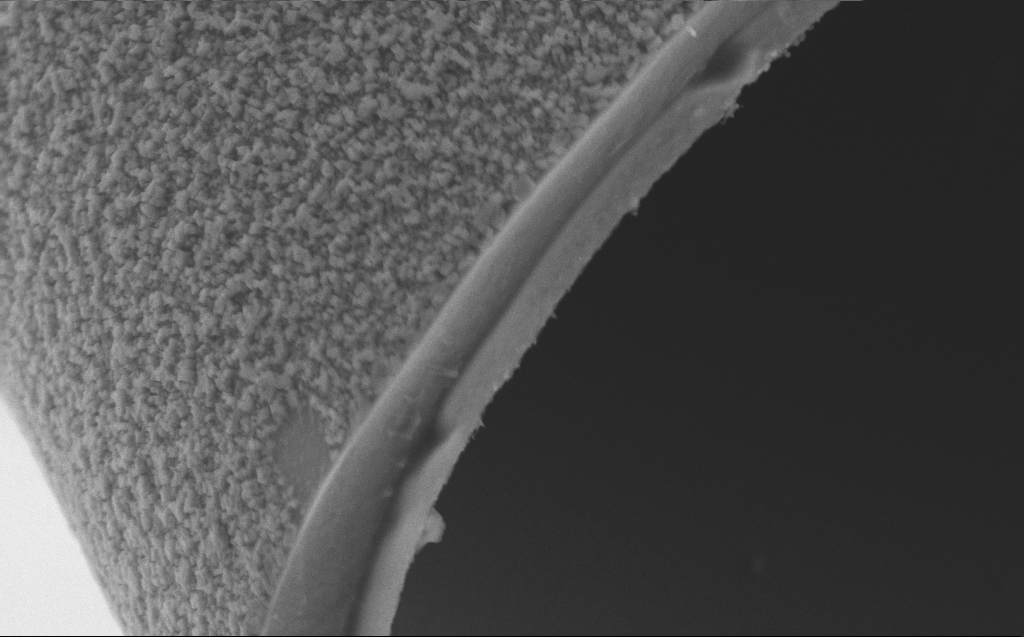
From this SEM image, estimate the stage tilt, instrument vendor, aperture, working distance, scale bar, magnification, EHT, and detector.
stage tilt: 45°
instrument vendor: Zeiss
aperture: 30 µm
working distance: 4 mm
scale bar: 2000 nm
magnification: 21.35 K X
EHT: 5 kV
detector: SE2